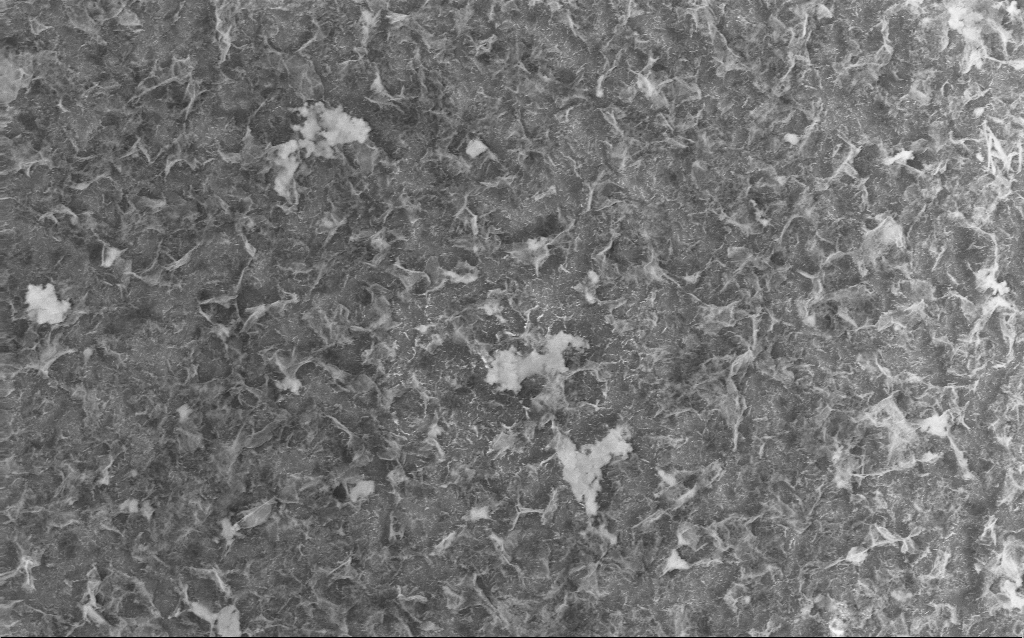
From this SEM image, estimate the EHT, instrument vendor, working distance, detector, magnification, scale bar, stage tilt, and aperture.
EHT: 10 kV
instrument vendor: Zeiss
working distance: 2.5 mm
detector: InLens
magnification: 10 K X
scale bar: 2000 nm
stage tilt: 0°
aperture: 30 µm